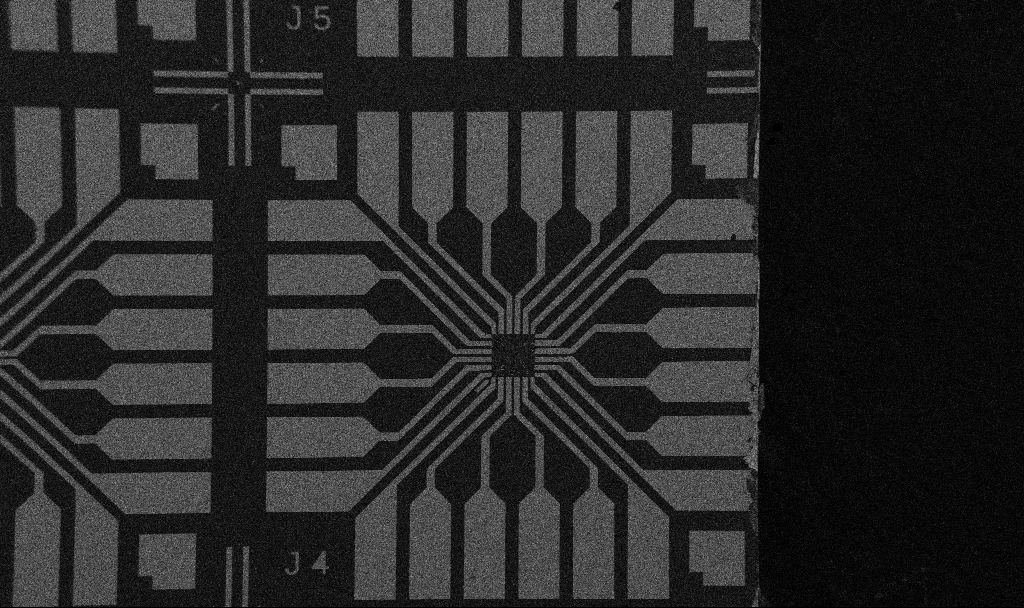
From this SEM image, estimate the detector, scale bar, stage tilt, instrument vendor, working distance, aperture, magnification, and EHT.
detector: SE2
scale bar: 200000 nm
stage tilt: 0°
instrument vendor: Zeiss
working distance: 10.7 mm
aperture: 30 µm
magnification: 0.1 K X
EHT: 5 kV